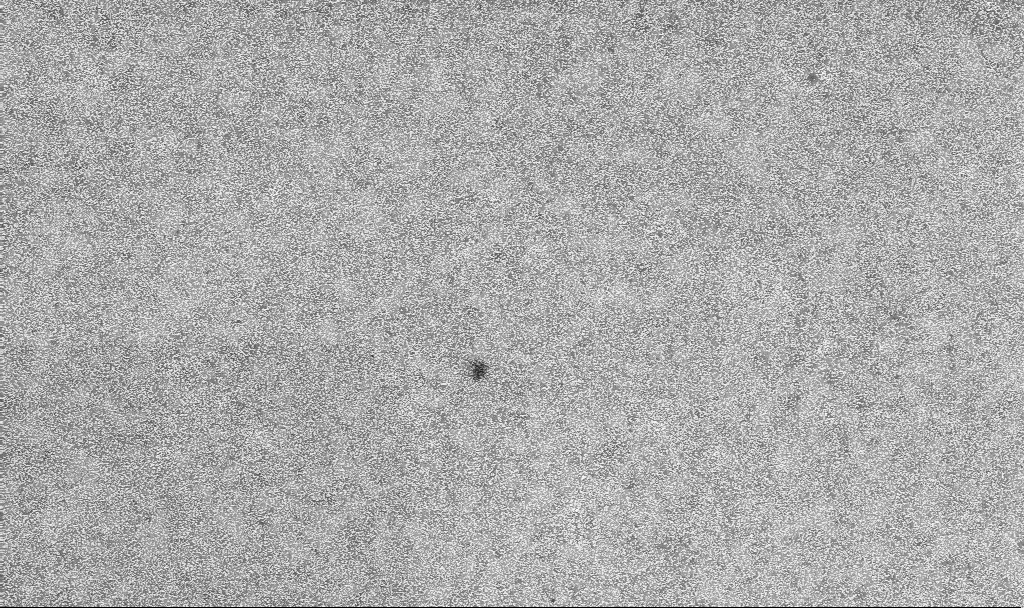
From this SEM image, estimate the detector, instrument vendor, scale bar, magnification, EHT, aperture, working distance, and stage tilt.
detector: InLens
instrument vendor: Zeiss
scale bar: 1000 nm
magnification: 20 K X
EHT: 10 kV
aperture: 30 µm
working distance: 3.3 mm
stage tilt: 0°